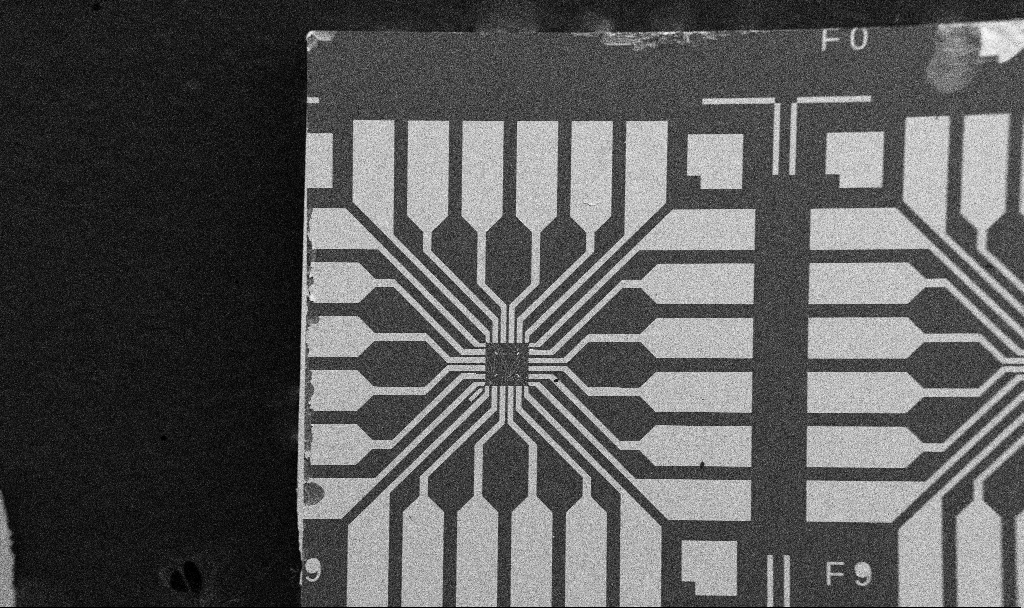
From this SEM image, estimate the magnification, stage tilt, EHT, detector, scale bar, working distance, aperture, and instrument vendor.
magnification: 0.1 K X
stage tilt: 0°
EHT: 5 kV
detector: SE2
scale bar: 200000 nm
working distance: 10.7 mm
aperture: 30 µm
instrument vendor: Zeiss